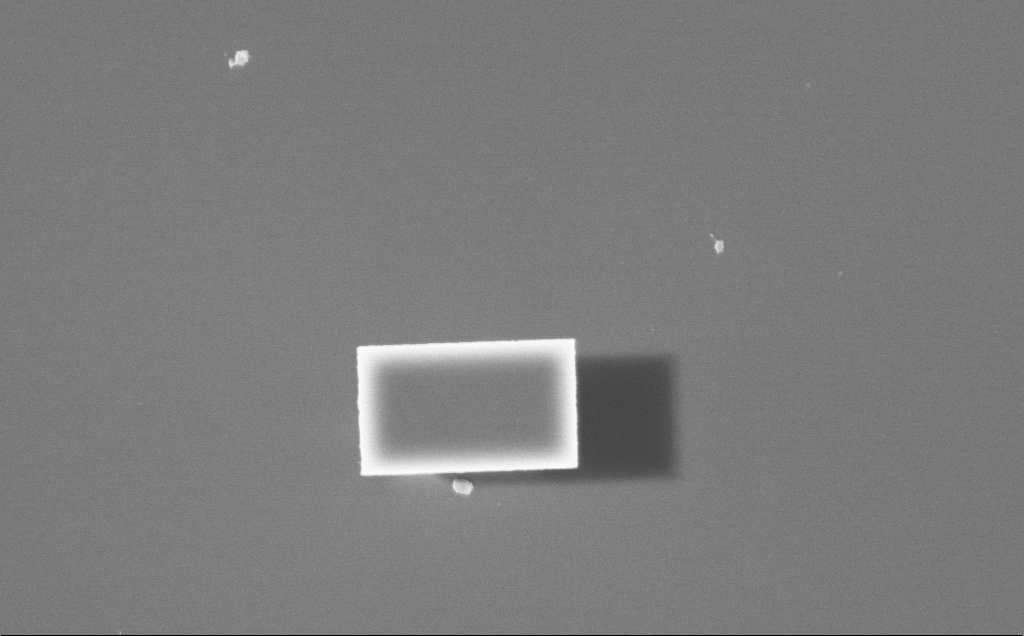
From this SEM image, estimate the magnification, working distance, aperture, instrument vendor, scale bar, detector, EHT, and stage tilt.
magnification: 16.61 K X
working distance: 4 mm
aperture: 30 µm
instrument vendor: Zeiss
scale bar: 2000 nm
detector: InLens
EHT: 7.5 kV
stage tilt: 0°